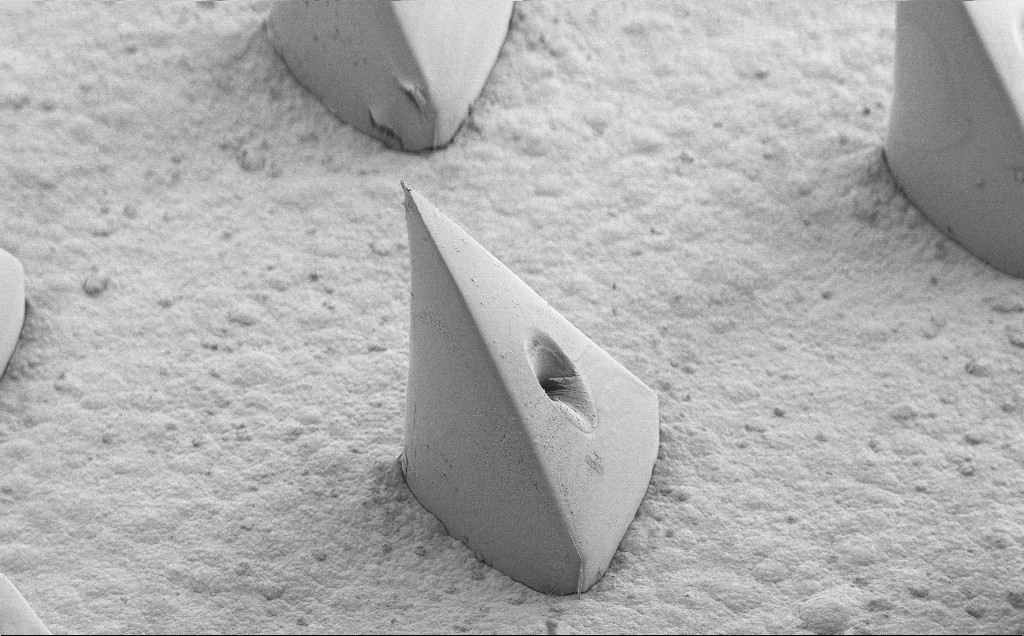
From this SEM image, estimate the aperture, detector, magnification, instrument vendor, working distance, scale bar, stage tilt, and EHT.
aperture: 30 µm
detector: SE2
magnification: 0.165 K X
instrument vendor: Zeiss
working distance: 8 mm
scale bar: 100000 nm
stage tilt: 40°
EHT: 5 kV